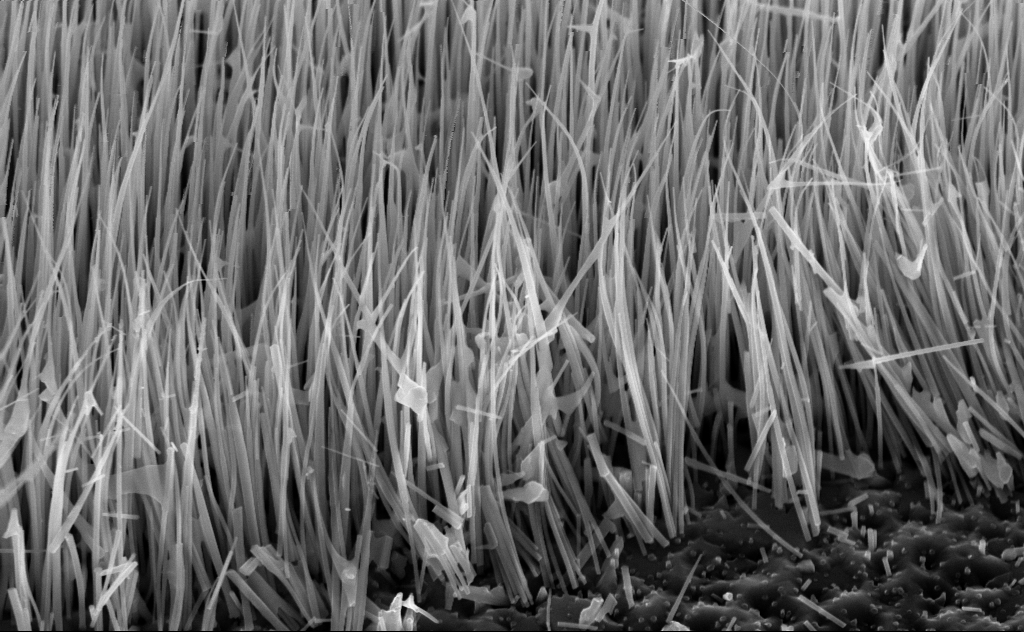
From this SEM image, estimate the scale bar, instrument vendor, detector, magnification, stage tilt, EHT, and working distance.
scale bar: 1000 nm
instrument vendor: Zeiss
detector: InLens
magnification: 44.89 K X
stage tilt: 45°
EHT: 10 kV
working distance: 6 mm